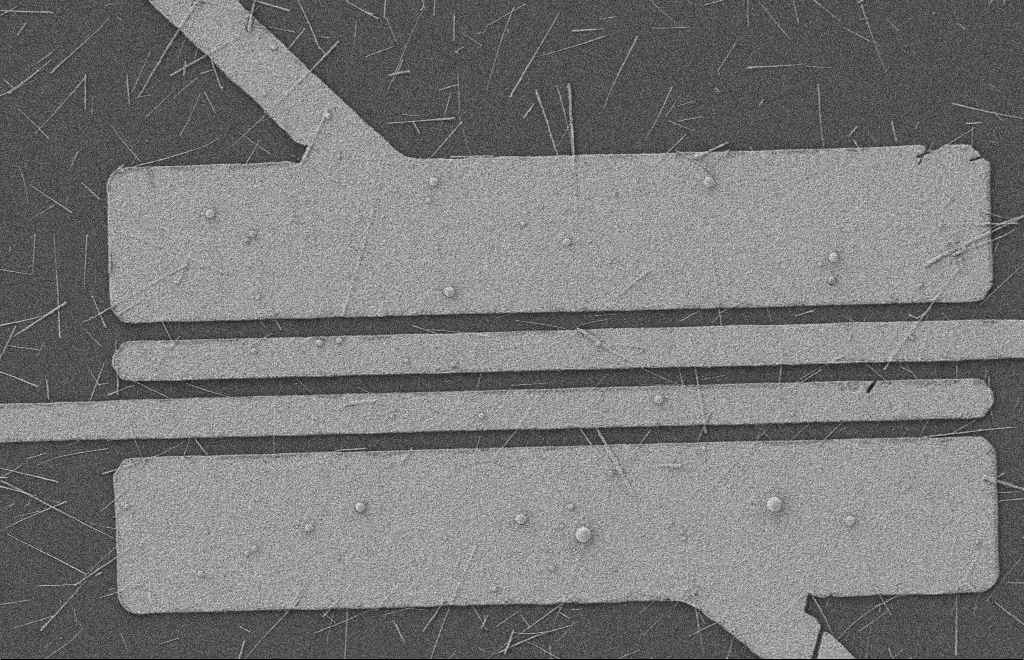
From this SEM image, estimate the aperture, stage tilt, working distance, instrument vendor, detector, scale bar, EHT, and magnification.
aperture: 20 µm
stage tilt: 0°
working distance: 8 mm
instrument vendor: Zeiss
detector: SE2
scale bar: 2000 nm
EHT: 2 kV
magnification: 5.31 K X